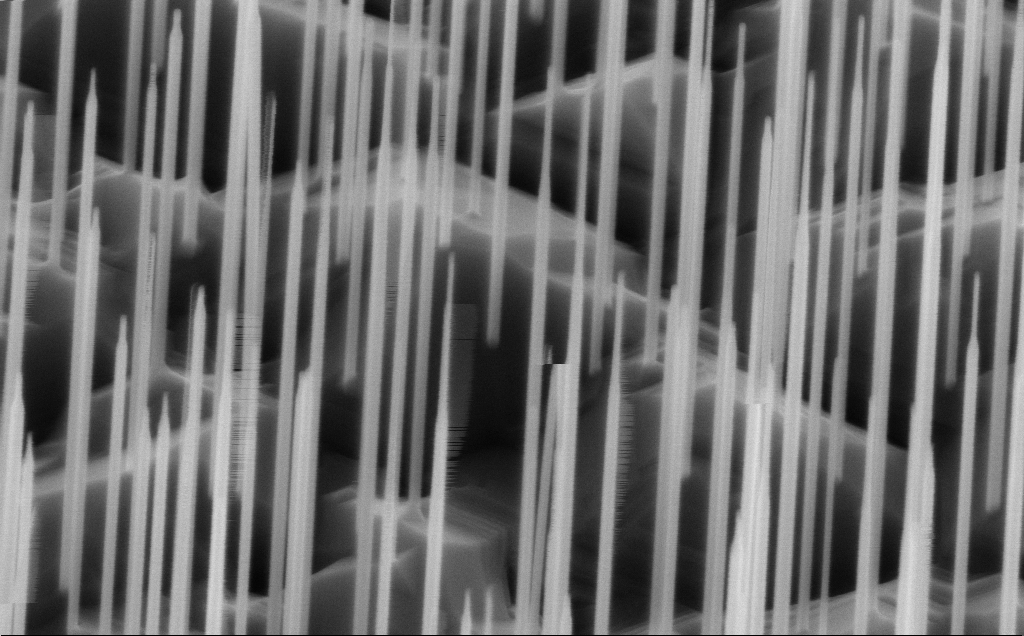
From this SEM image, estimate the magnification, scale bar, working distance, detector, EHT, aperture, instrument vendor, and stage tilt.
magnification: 80 K X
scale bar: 200 nm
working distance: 5 mm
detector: InLens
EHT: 10 kV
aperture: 30 µm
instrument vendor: Zeiss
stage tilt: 45°